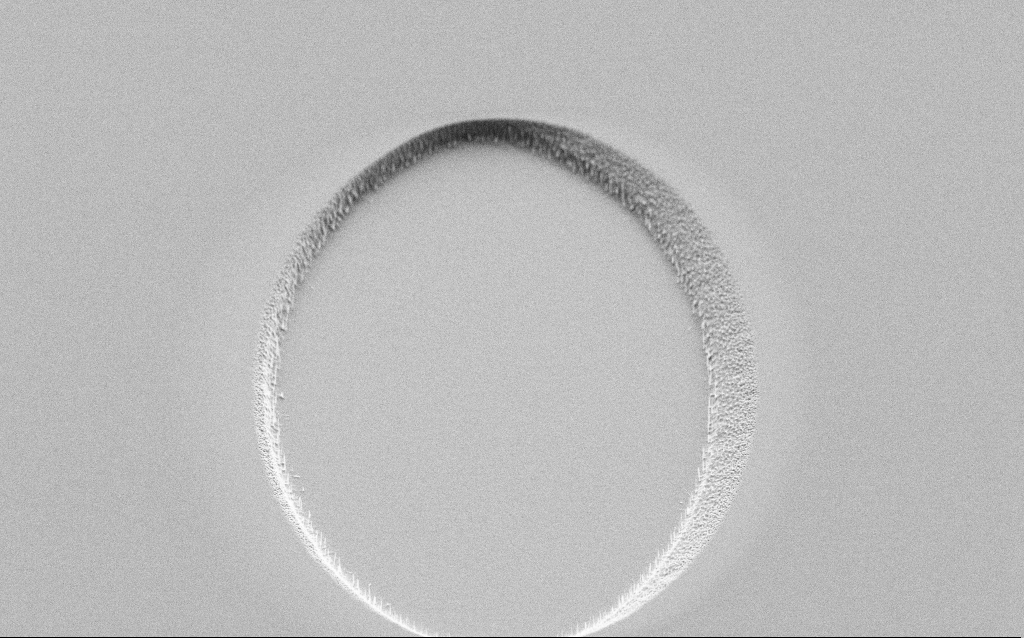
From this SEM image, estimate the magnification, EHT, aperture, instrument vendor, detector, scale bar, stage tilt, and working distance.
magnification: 5.05 K X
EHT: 3 kV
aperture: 30 µm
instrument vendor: Zeiss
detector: SE2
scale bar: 10000 nm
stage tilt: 45°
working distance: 6 mm